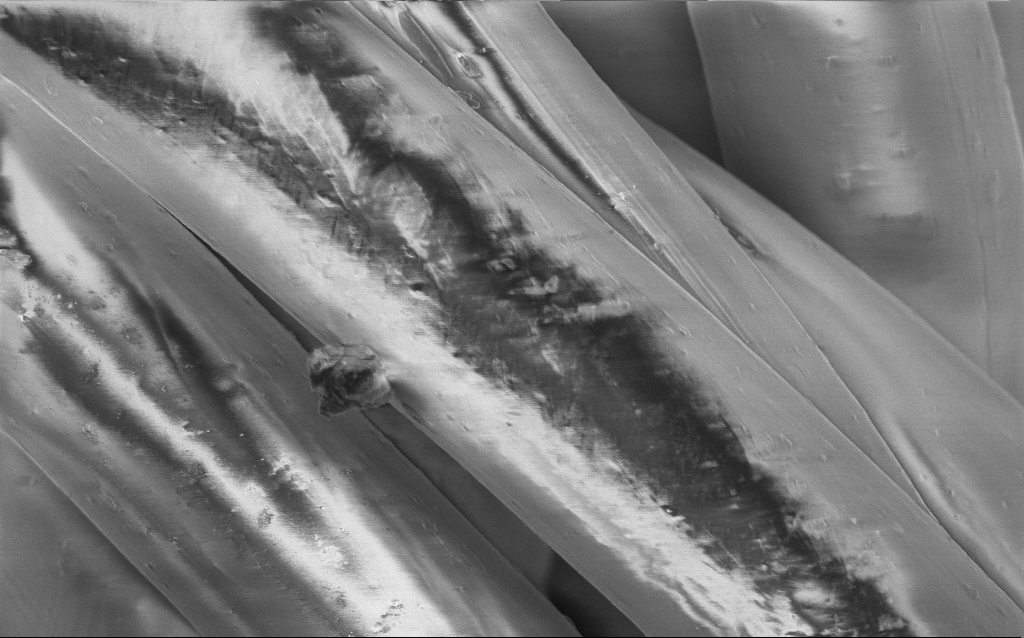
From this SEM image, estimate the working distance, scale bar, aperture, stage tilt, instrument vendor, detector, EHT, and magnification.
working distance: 5 mm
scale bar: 10000 nm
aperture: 30 µm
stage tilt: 0°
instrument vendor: Zeiss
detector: InLens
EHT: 2 kV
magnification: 4.69 K X